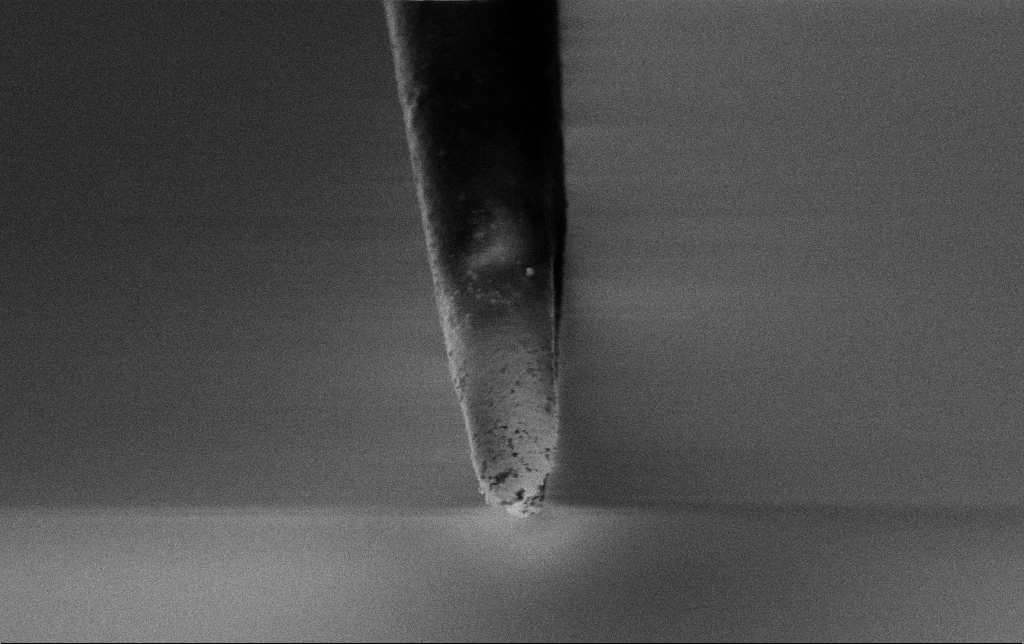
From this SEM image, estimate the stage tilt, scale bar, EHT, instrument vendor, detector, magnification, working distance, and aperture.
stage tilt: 45°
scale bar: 1000 nm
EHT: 3 kV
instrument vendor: Zeiss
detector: InLens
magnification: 25 K X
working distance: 7.4 mm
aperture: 30 µm